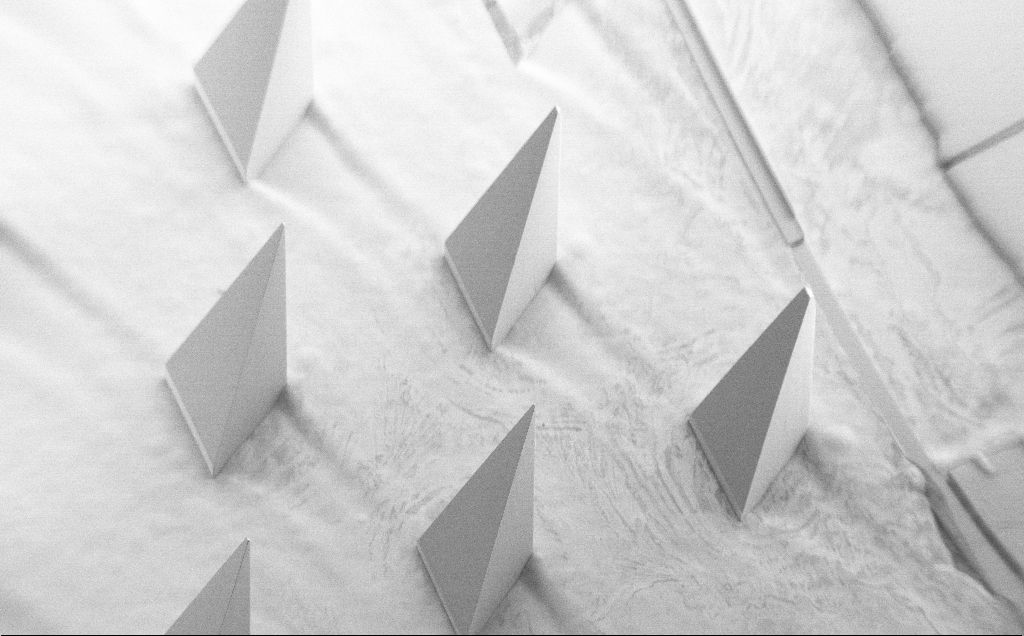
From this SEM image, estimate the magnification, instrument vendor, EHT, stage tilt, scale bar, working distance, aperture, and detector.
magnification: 0.071 K X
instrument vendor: Zeiss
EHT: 5 kV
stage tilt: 40°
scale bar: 1e+06 nm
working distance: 8 mm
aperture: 30 µm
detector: SE2